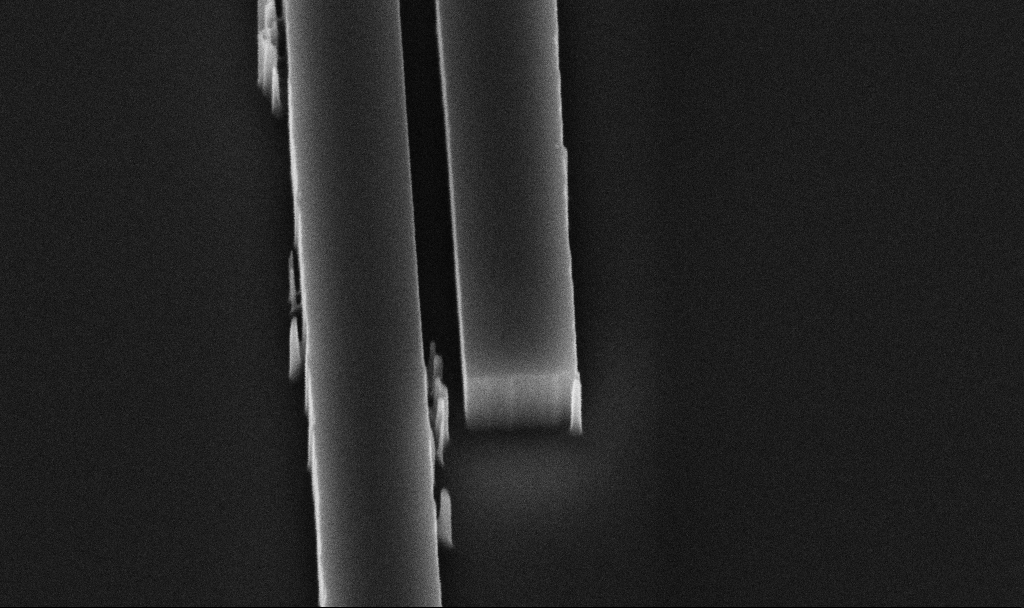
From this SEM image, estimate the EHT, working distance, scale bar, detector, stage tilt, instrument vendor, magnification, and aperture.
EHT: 5 kV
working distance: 10 mm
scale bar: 200 nm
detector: InLens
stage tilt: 45°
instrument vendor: Zeiss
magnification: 85.28 K X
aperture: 30 µm